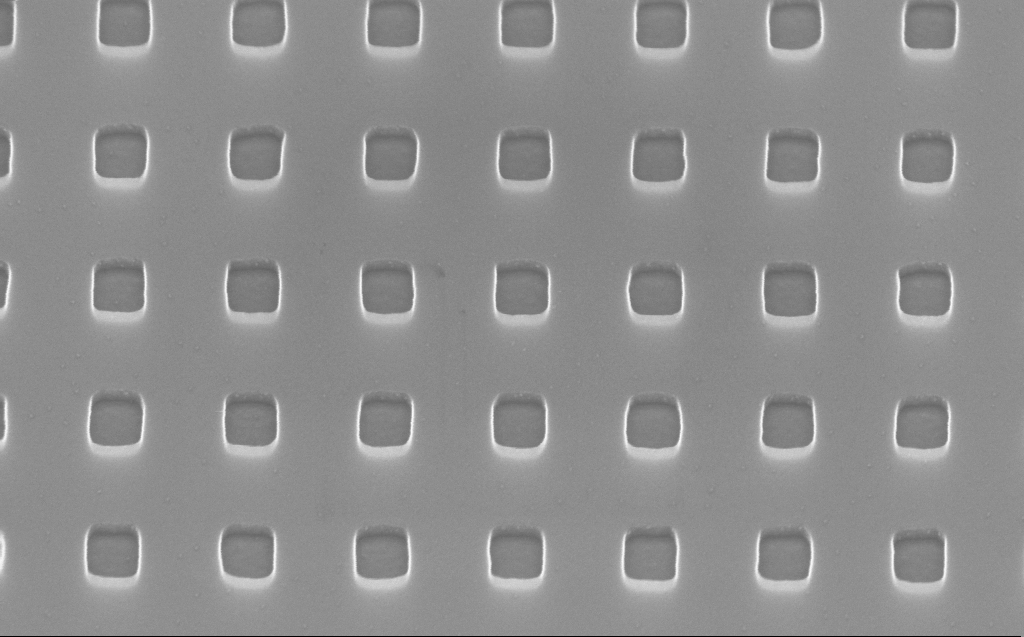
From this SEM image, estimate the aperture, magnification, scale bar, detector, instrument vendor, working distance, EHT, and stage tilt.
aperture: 30 µm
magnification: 100 K X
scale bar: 200 nm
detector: InLens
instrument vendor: Zeiss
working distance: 5 mm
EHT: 10 kV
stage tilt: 45°